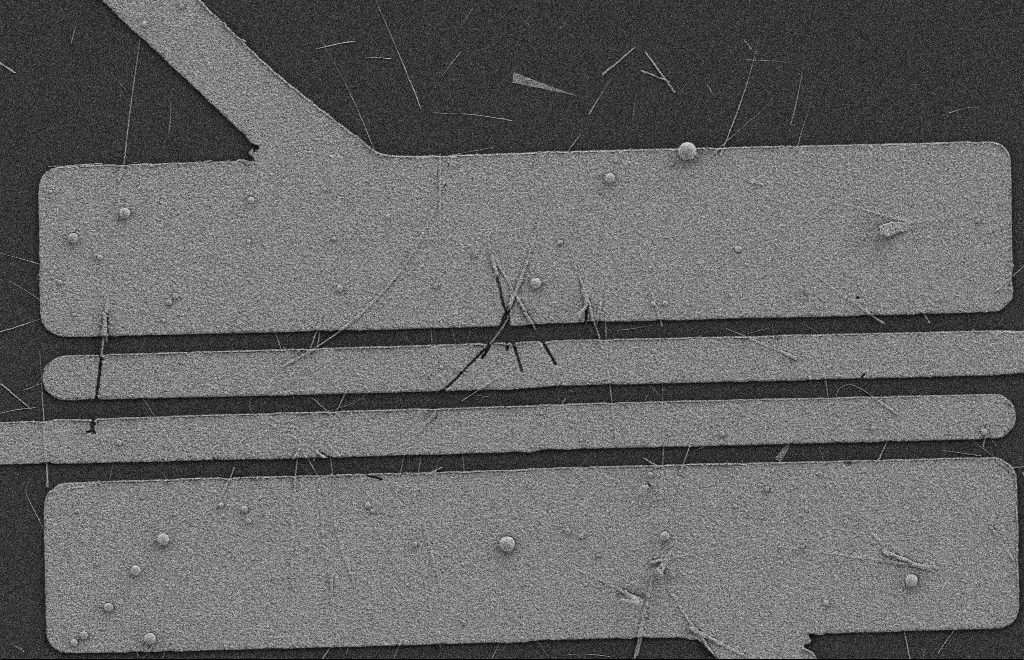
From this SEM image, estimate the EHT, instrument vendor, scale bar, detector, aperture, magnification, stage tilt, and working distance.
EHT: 2 kV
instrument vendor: Zeiss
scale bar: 2000 nm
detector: SE2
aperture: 20 µm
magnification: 5.83 K X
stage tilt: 0°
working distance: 9 mm